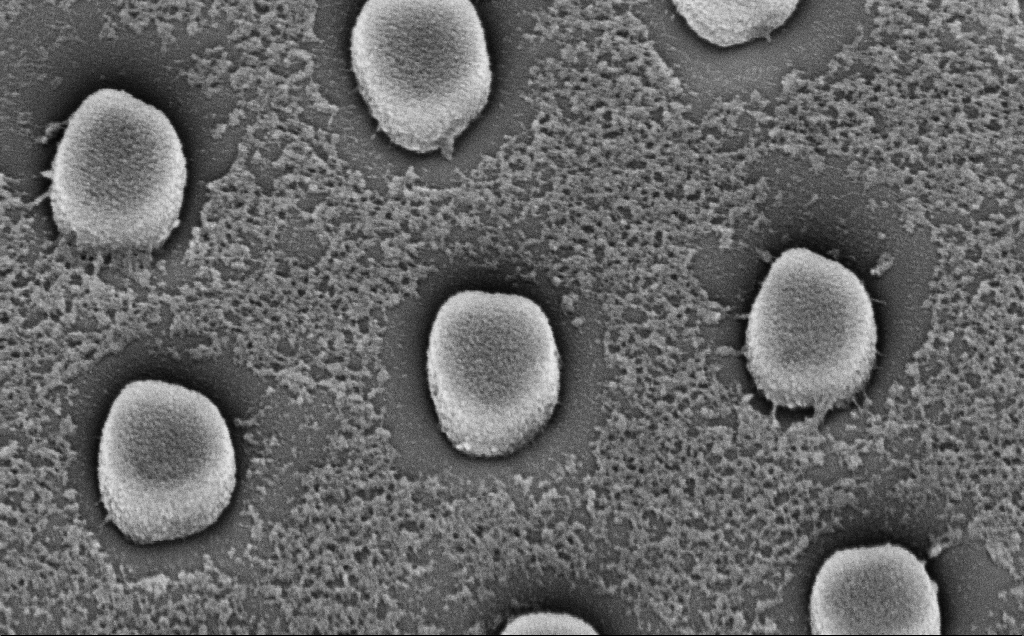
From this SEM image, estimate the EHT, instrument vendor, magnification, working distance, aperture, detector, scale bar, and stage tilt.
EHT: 5 kV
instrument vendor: Zeiss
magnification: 80.72 K X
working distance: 7 mm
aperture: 30 µm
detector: InLens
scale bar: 200 nm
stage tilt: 45°